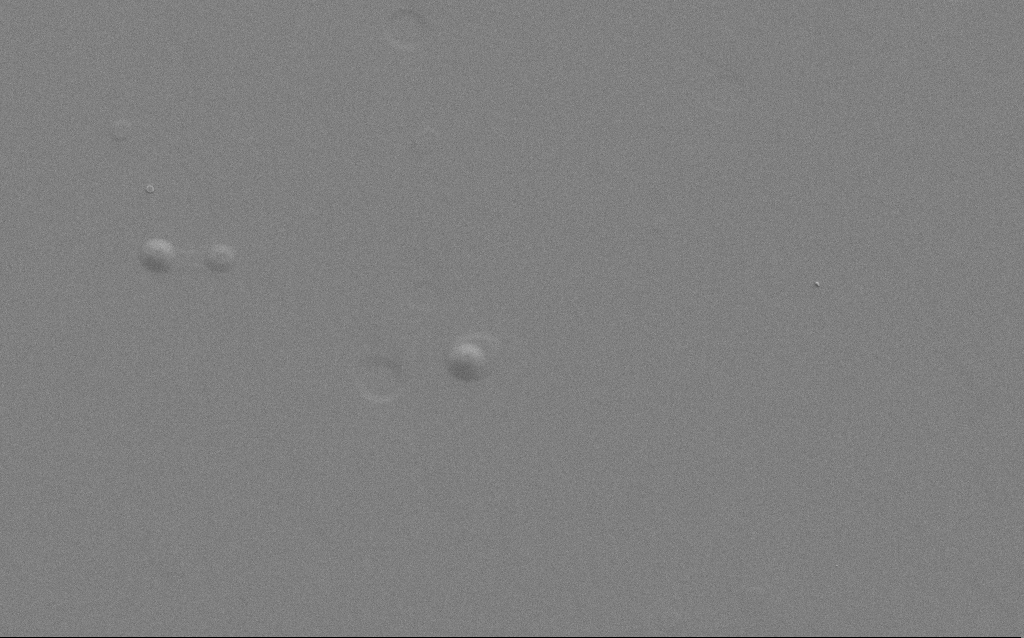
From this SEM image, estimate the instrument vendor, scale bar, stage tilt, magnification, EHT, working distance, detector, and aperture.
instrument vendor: Zeiss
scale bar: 2000 nm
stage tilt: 20°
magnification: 10.46 K X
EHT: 5 kV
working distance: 4 mm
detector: SE2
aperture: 30 µm